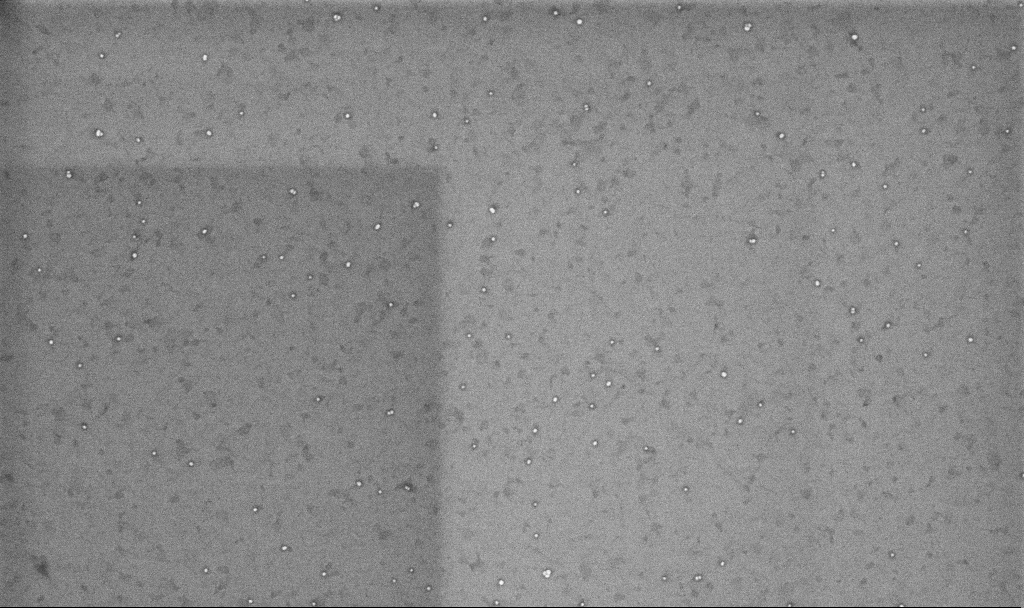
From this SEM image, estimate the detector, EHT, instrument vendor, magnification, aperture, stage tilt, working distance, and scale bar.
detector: InLens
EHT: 10 kV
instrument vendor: Zeiss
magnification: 50.38 K X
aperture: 30 µm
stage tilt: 0°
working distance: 3.3 mm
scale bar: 1000 nm